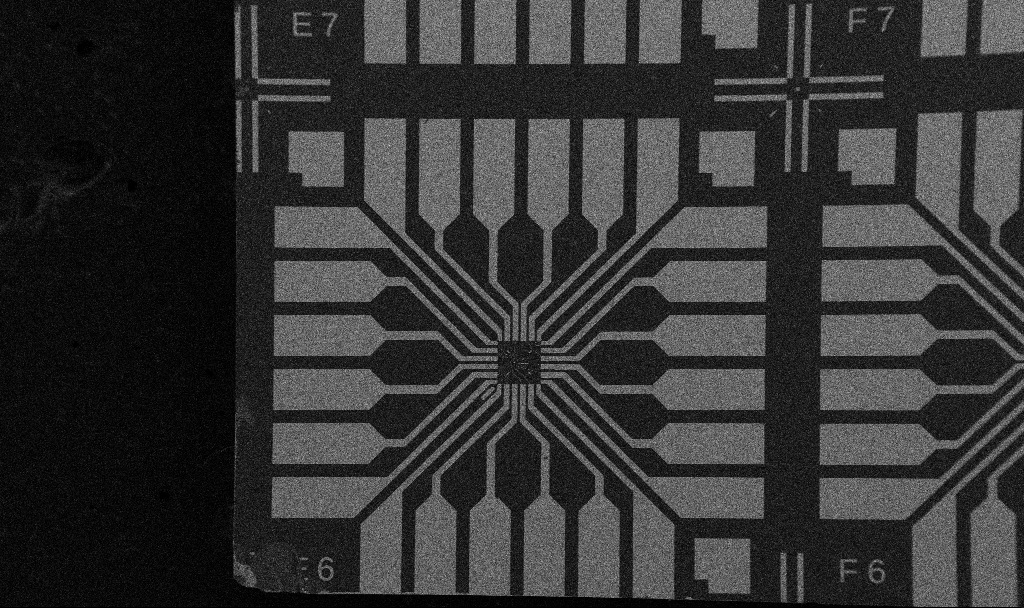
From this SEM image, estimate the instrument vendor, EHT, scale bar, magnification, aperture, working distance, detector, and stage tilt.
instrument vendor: Zeiss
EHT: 5 kV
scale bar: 200000 nm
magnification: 0.1 K X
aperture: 30 µm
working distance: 10.7 mm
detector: SE2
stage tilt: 0°